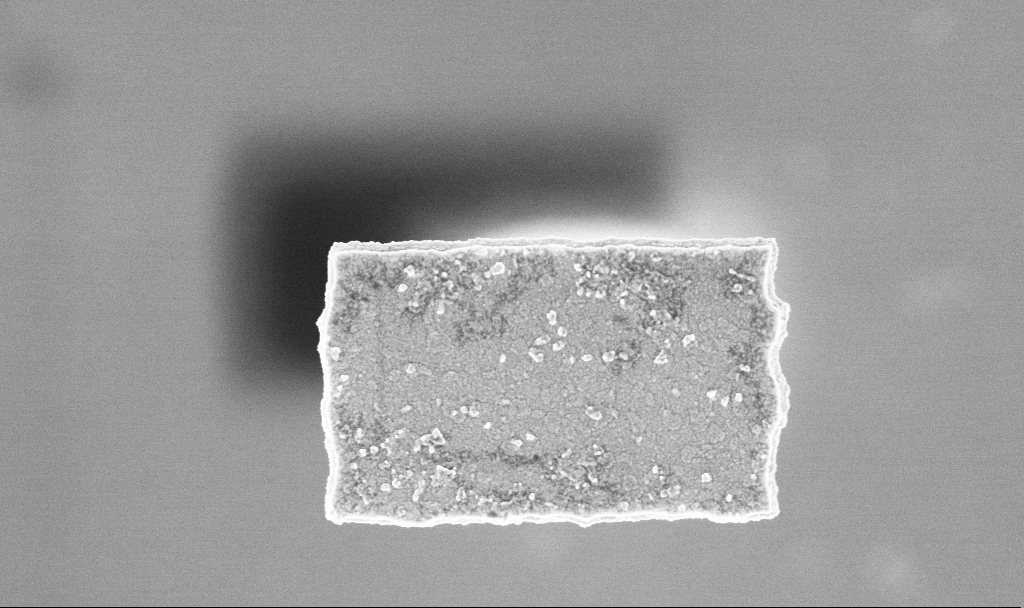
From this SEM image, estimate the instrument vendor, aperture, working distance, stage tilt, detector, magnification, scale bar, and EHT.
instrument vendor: Zeiss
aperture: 30 µm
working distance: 3.2 mm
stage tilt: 0°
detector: InLens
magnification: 57.17 K X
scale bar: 1000 nm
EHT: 3 kV